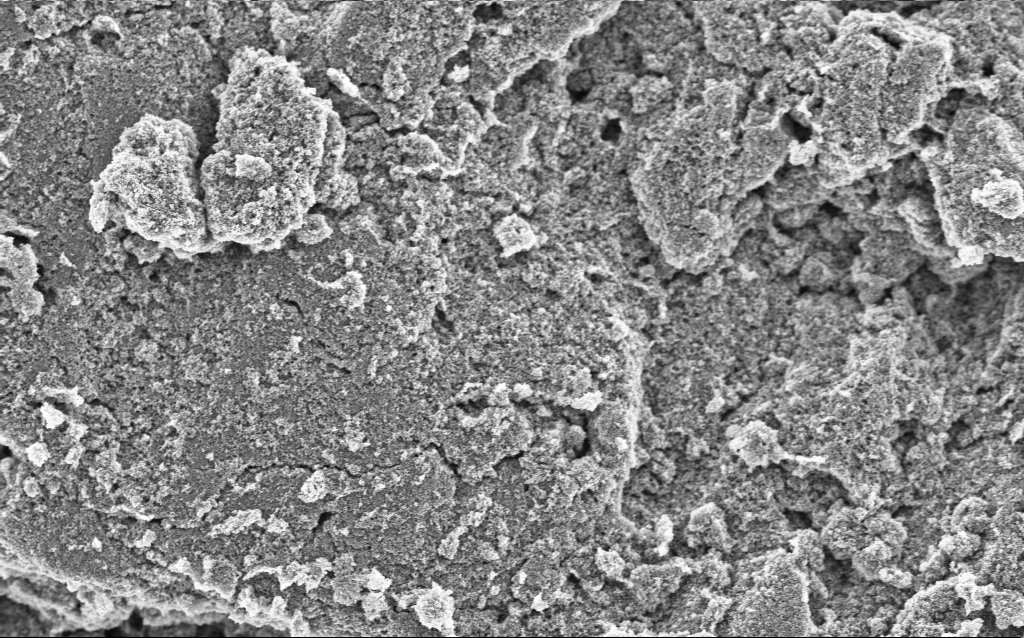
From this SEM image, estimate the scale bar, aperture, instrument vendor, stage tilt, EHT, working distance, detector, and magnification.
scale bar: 10000 nm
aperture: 30 µm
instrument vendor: Zeiss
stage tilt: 0°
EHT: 5 kV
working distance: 4.4 mm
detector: InLens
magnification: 6.42 K X